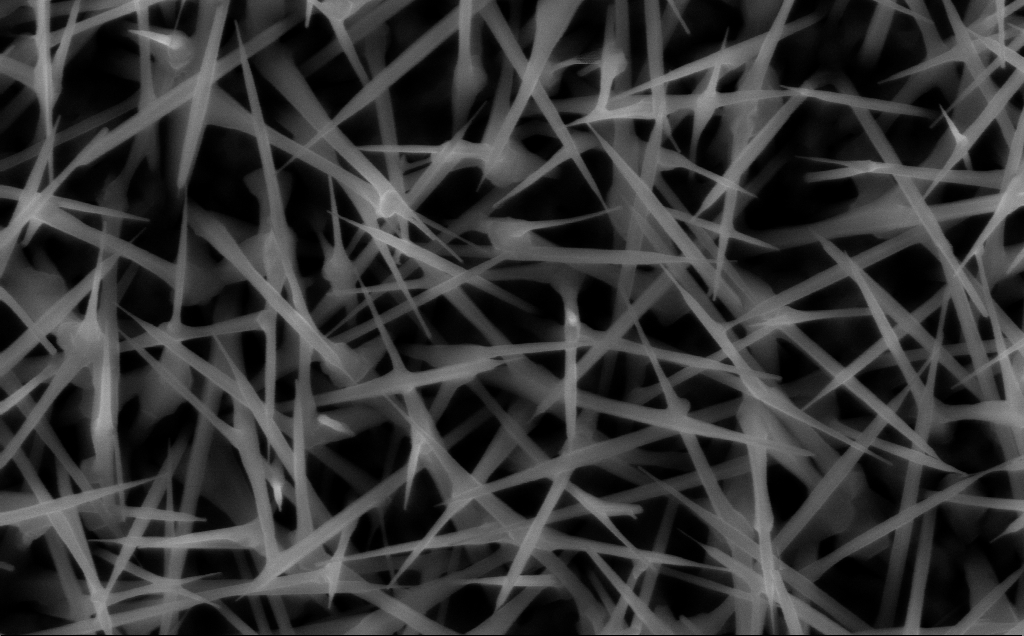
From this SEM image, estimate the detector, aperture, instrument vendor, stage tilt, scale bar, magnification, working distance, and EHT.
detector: InLens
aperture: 30 µm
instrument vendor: Zeiss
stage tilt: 0°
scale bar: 200 nm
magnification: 80 K X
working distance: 7 mm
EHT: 10 kV